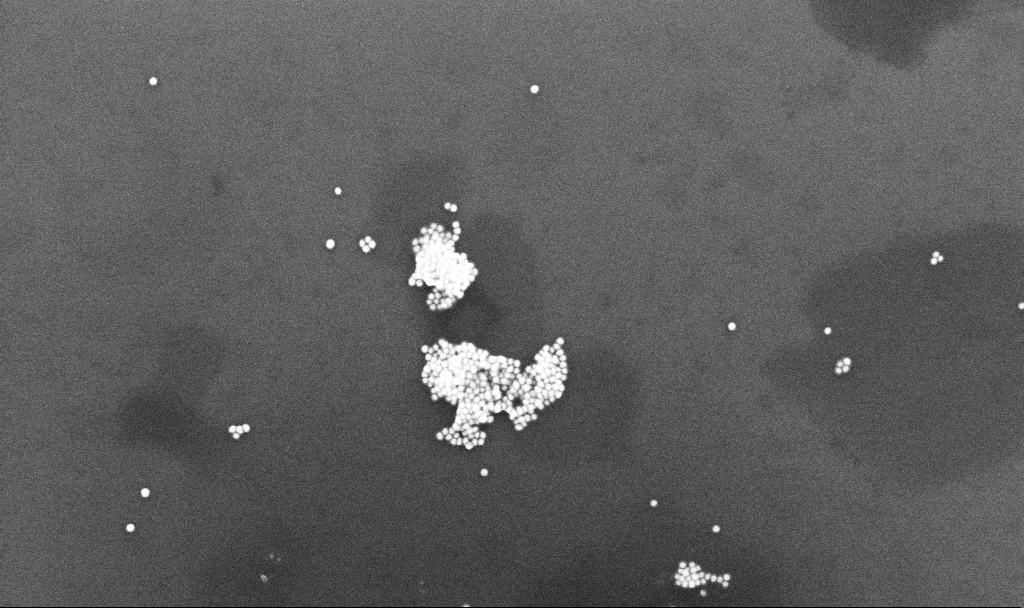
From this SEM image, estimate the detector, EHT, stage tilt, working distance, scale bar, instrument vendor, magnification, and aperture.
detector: InLens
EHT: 10 kV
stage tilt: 0°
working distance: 3.3 mm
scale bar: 200 nm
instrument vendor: Zeiss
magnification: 108.67 K X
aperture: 30 µm